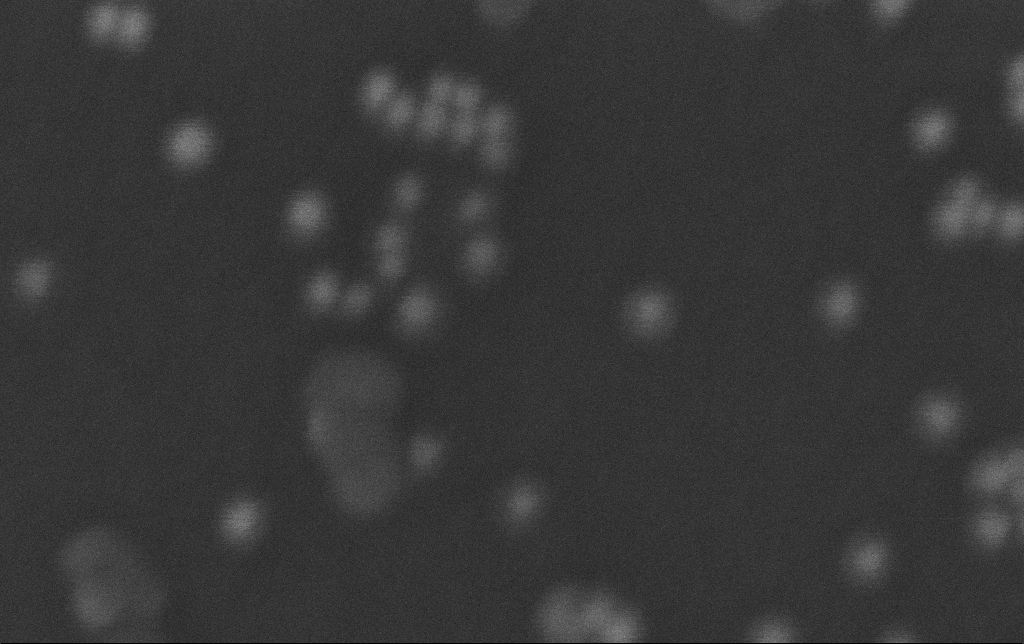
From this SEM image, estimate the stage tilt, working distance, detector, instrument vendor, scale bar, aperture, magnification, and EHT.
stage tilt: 0°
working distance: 3.2 mm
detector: InLens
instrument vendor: Zeiss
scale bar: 20 nm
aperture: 30 µm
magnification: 905.76 K X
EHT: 3 kV